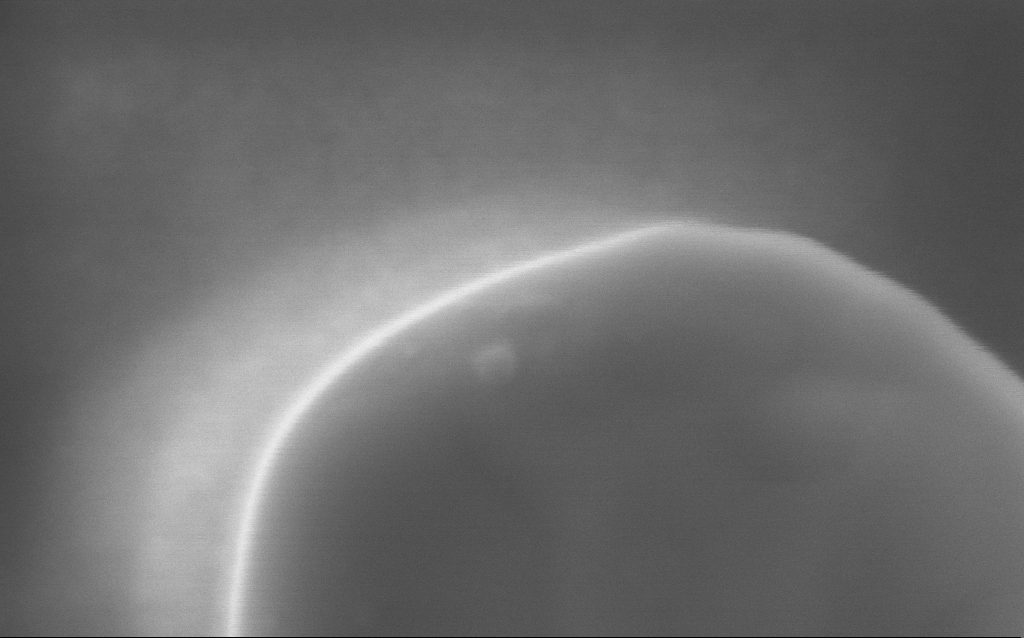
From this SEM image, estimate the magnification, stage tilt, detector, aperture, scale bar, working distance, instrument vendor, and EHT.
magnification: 350 K X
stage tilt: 0°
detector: InLens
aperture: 30 µm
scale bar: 100 nm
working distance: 3 mm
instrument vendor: Zeiss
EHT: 5 kV